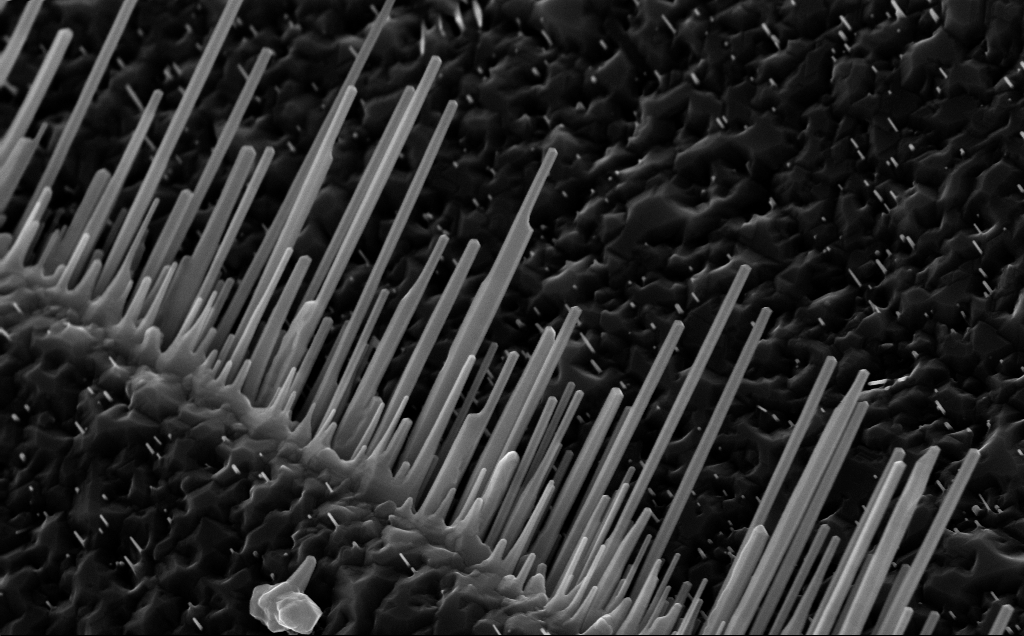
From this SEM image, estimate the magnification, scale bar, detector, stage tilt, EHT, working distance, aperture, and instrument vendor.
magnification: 56.97 K X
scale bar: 1000 nm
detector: InLens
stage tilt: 0°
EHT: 10 kV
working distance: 5 mm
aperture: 30 µm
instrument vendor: Zeiss